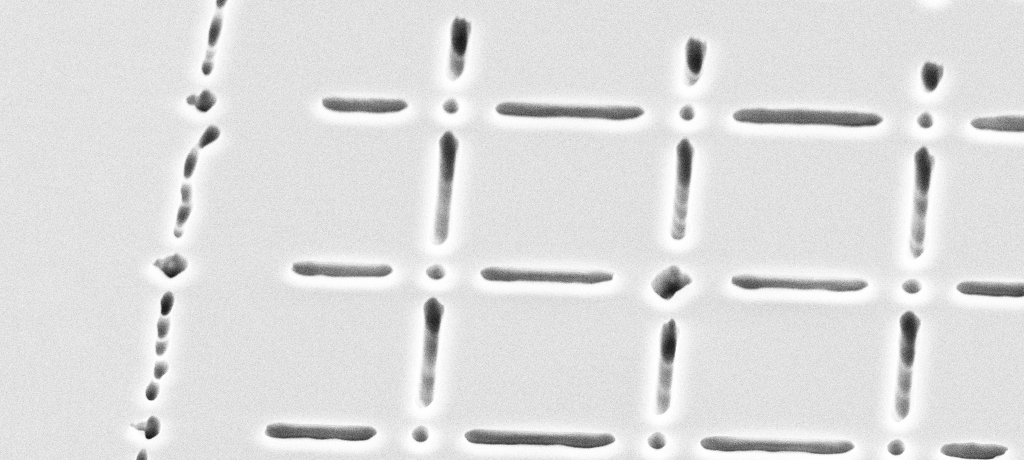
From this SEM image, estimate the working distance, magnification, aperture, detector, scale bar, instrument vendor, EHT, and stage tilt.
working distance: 13 mm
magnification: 4.29 K X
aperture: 30 µm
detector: SE2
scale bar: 10000 nm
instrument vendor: Zeiss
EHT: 10 kV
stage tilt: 45°